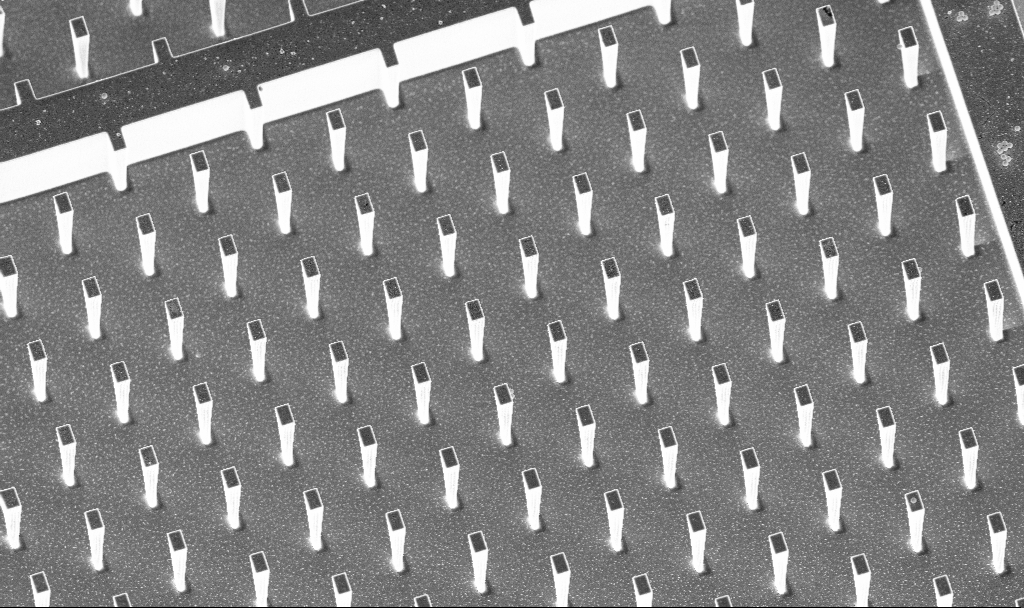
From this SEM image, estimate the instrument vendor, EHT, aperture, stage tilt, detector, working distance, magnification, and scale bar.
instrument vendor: Zeiss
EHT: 5 kV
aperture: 30 µm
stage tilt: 20°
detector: InLens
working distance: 5.2 mm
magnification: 2.84 K X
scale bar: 10000 nm